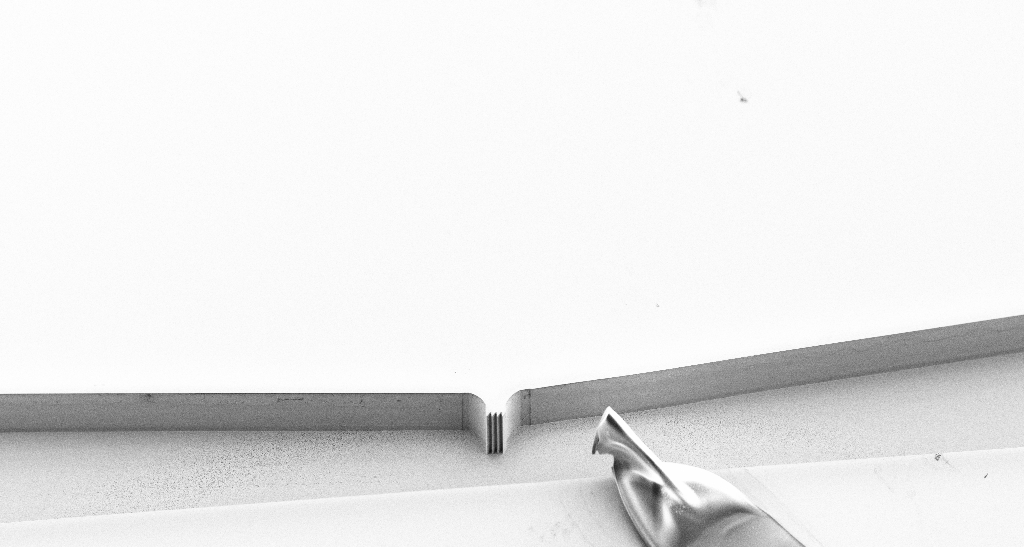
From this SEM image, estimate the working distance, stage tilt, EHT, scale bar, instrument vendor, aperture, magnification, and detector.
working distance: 7 mm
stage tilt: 45°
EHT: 5 kV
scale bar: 100000 nm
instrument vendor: Zeiss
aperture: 30 µm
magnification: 0.173 K X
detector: SE2